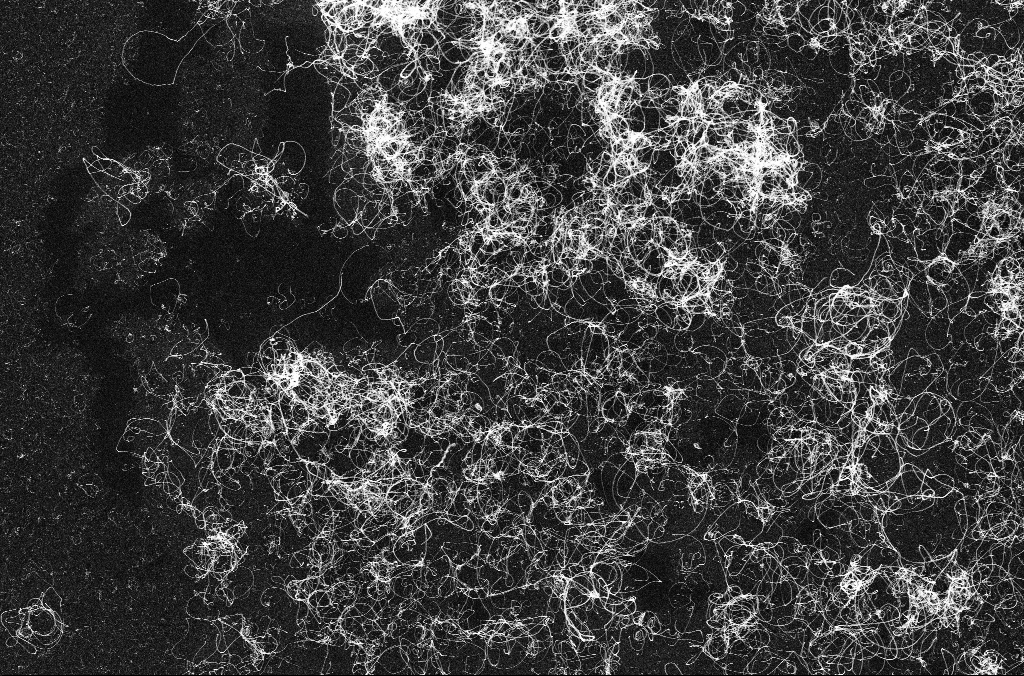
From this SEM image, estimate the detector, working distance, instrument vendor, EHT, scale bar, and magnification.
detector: InLens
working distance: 3.3 mm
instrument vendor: Zeiss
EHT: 10 kV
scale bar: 2000 nm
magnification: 10 K X